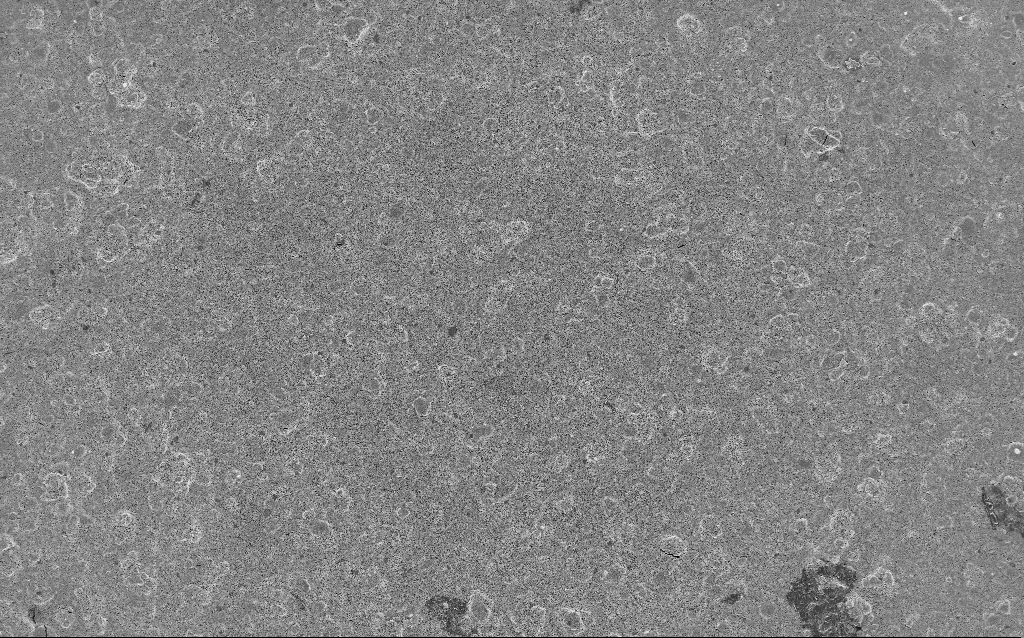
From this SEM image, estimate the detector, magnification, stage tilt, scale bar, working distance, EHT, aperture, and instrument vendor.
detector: InLens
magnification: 0.77 K X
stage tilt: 0°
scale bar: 20000 nm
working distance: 7.5 mm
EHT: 3 kV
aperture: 30 µm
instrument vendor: Zeiss